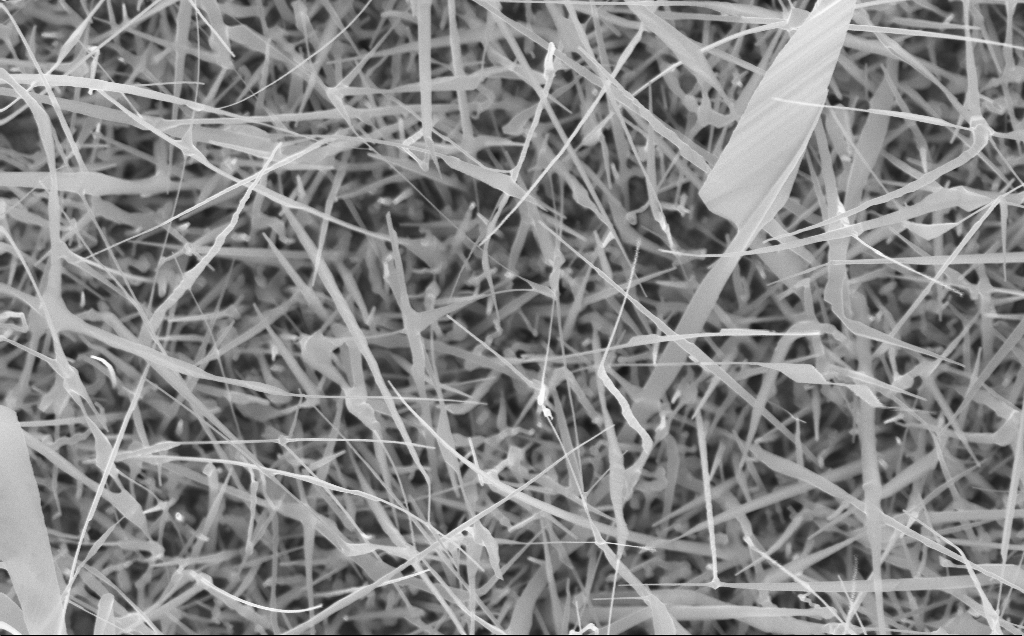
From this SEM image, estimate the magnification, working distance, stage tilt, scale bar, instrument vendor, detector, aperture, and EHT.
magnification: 33.65 K X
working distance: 4 mm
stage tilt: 0°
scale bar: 1000 nm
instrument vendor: Zeiss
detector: InLens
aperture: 30 µm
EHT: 10 kV